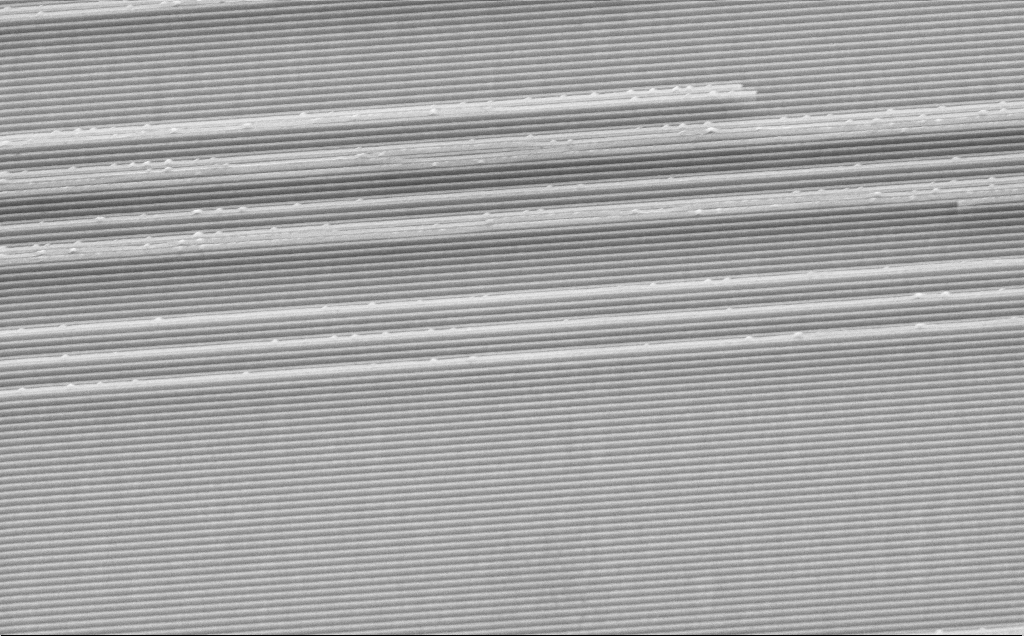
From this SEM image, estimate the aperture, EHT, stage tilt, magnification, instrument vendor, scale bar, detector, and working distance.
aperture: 30 µm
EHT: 10 kV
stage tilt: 35°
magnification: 10.86 K X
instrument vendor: Zeiss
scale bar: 2000 nm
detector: InLens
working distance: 4 mm